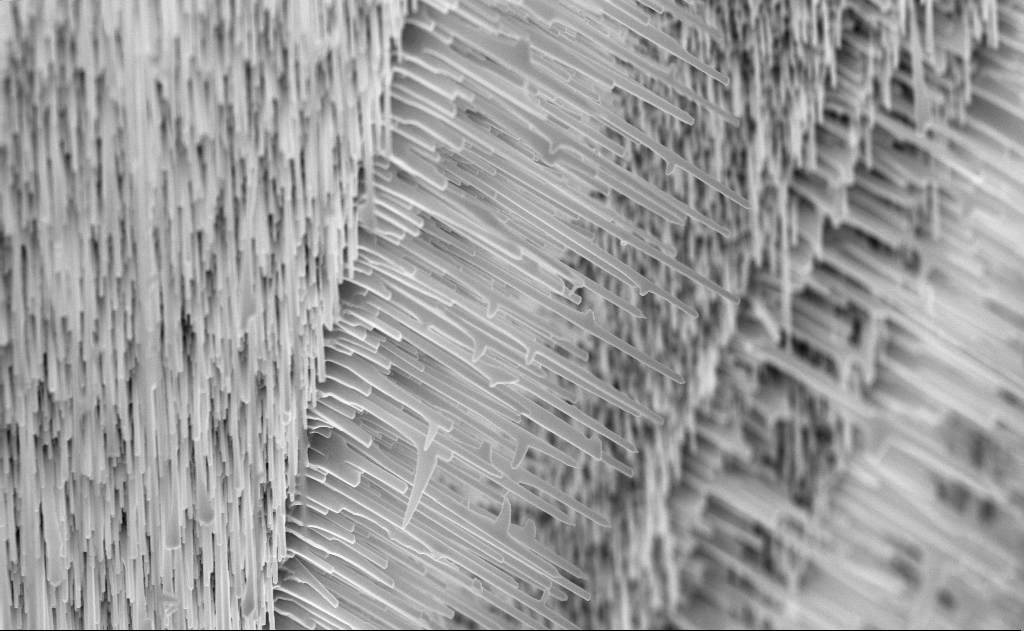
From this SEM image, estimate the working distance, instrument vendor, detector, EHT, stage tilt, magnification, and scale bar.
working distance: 6 mm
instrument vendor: Zeiss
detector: InLens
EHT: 10 kV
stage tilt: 0°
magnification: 20 K X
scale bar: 2000 nm